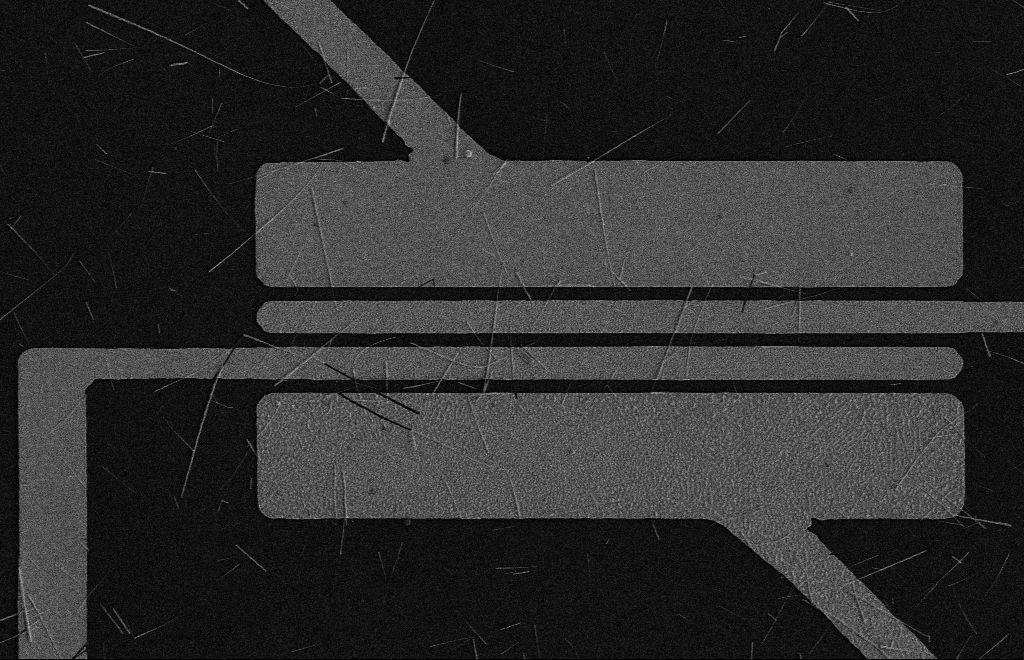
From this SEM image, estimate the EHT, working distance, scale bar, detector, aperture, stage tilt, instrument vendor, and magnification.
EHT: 5 kV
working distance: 16 mm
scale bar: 10000 nm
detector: SE2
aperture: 10 µm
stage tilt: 0°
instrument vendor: Zeiss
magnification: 4.28 K X